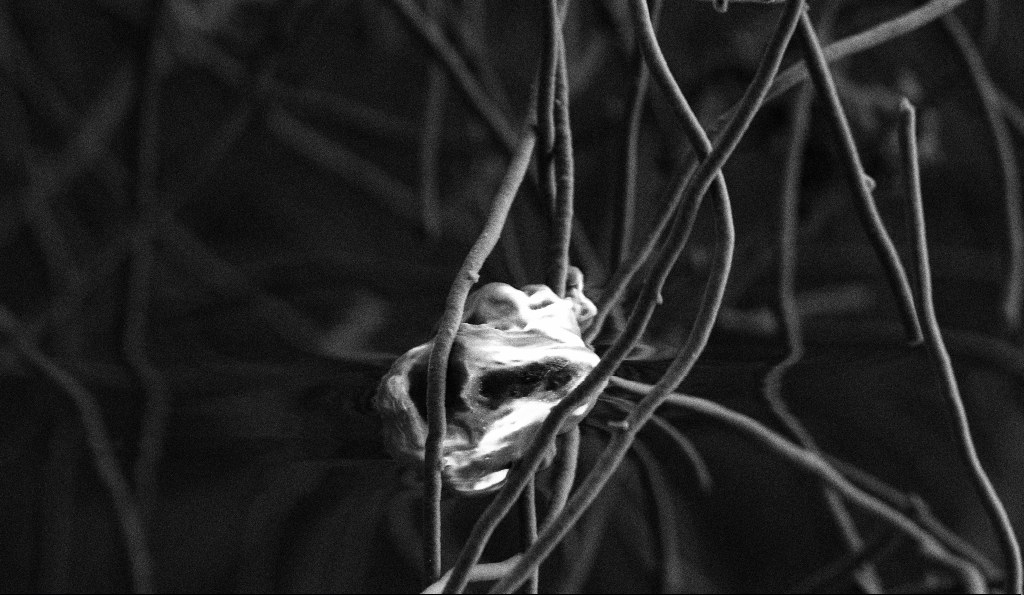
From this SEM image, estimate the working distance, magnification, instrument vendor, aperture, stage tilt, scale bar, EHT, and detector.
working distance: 5.4 mm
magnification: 11.17 K X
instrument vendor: Zeiss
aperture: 30 µm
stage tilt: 0°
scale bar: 2000 nm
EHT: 3 kV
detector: SE2